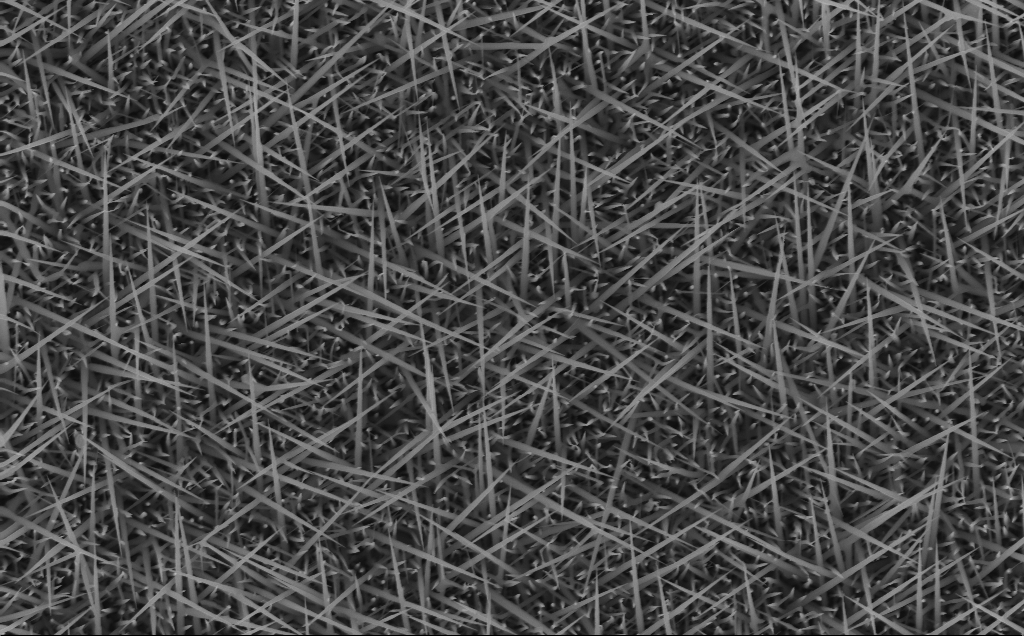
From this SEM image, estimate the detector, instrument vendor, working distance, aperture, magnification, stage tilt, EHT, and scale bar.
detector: InLens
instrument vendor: Zeiss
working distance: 6 mm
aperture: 30 µm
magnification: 40 K X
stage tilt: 0°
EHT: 10 kV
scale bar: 1000 nm